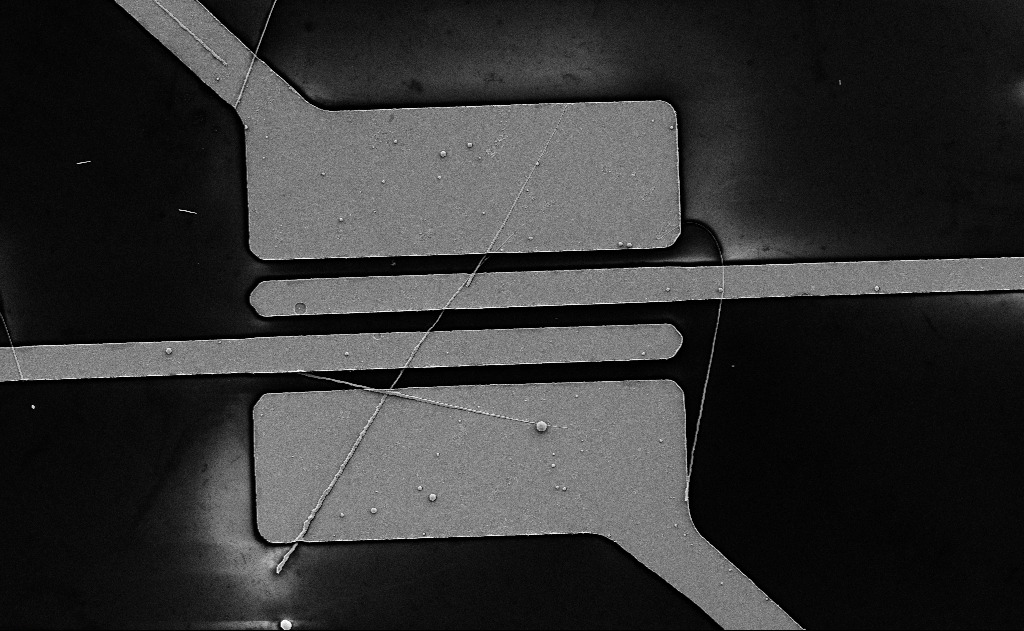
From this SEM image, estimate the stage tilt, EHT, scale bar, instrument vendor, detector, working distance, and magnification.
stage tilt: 0°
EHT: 5 kV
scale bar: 10000 nm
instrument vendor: Zeiss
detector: SE2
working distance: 18 mm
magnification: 5.21 K X